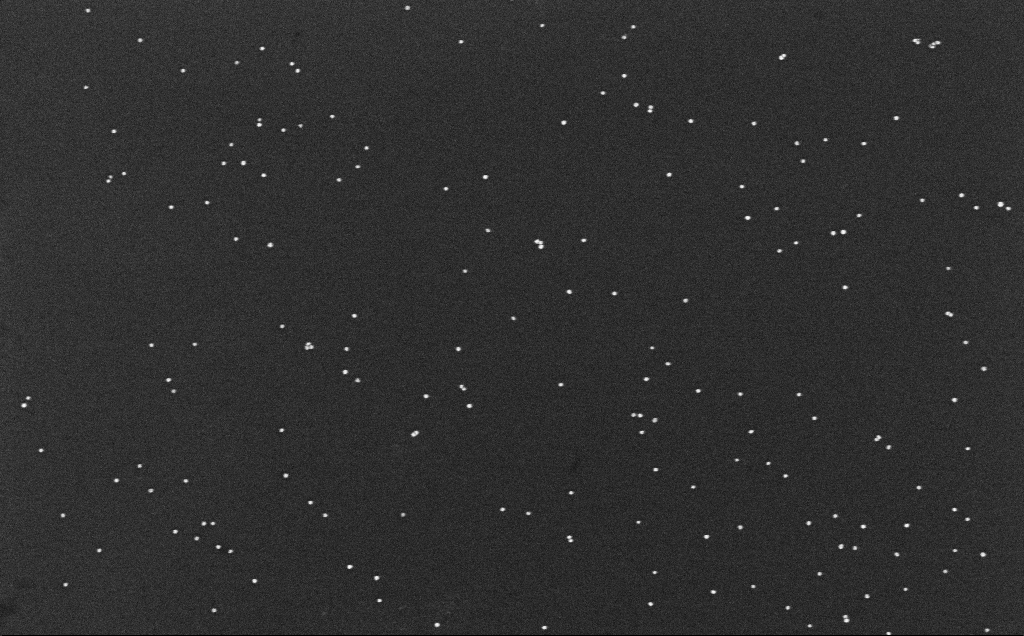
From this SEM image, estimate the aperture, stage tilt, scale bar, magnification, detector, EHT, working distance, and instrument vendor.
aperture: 30 µm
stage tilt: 0°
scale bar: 200 nm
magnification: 100 K X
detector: InLens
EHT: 10 kV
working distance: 6.6 mm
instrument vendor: Zeiss